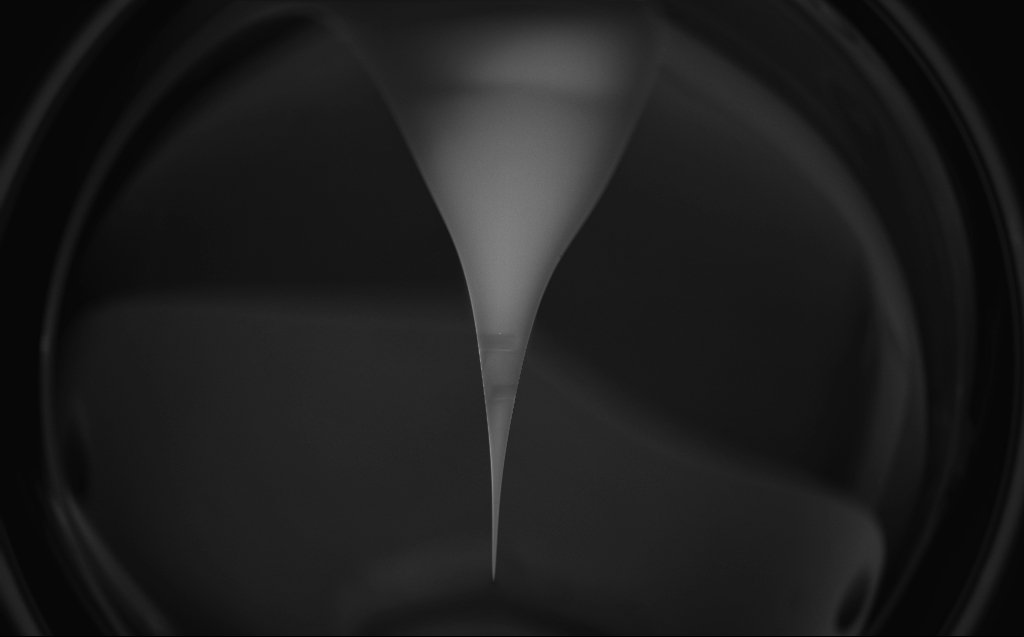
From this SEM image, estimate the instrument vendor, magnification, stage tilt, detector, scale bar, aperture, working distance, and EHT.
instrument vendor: Zeiss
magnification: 0.08 K X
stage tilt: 45.1°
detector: InLens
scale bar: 200000 nm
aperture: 30 µm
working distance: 3 mm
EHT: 0.75 kV